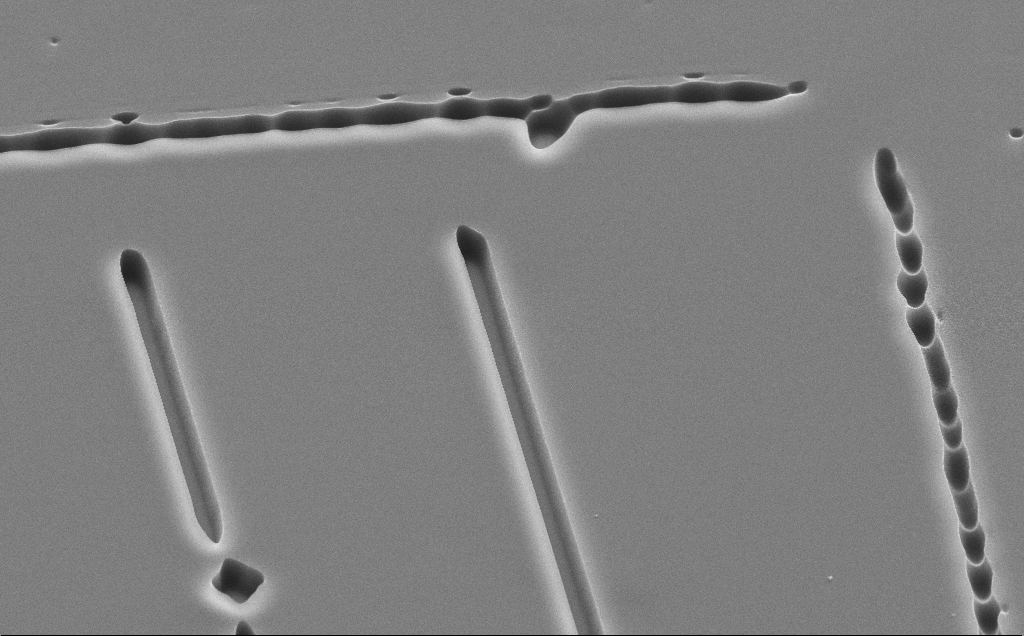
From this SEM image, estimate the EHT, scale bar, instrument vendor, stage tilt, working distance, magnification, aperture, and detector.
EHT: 10 kV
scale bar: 10000 nm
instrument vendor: Zeiss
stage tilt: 45°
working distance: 11 mm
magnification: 6.19 K X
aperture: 30 µm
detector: SE2